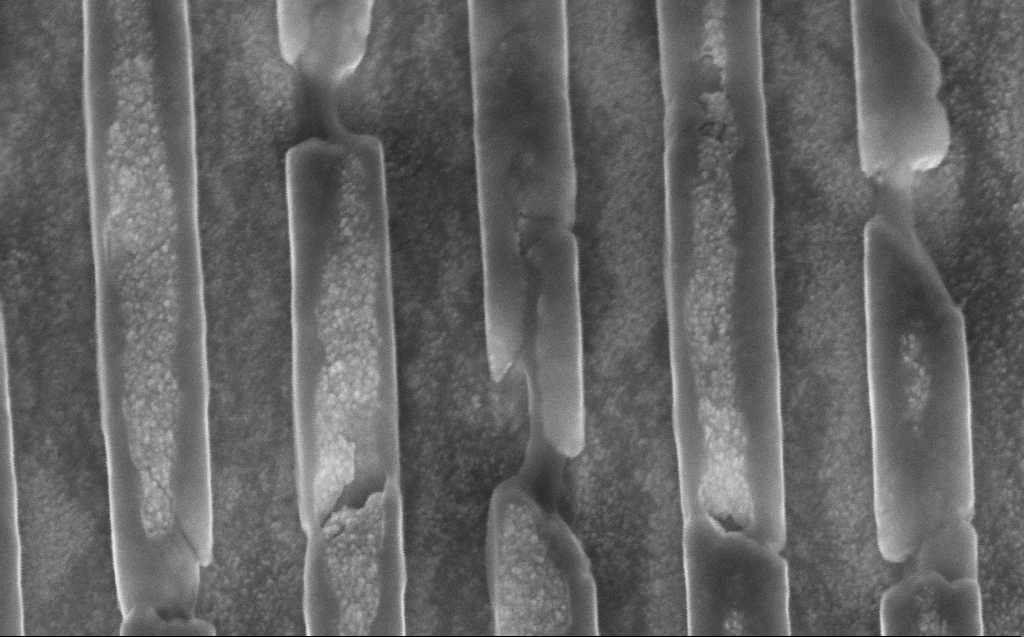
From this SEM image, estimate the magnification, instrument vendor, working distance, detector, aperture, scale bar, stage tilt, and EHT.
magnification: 141.46 K X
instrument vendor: Zeiss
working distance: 8 mm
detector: InLens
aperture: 30 µm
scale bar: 200 nm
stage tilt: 45°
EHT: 10 kV